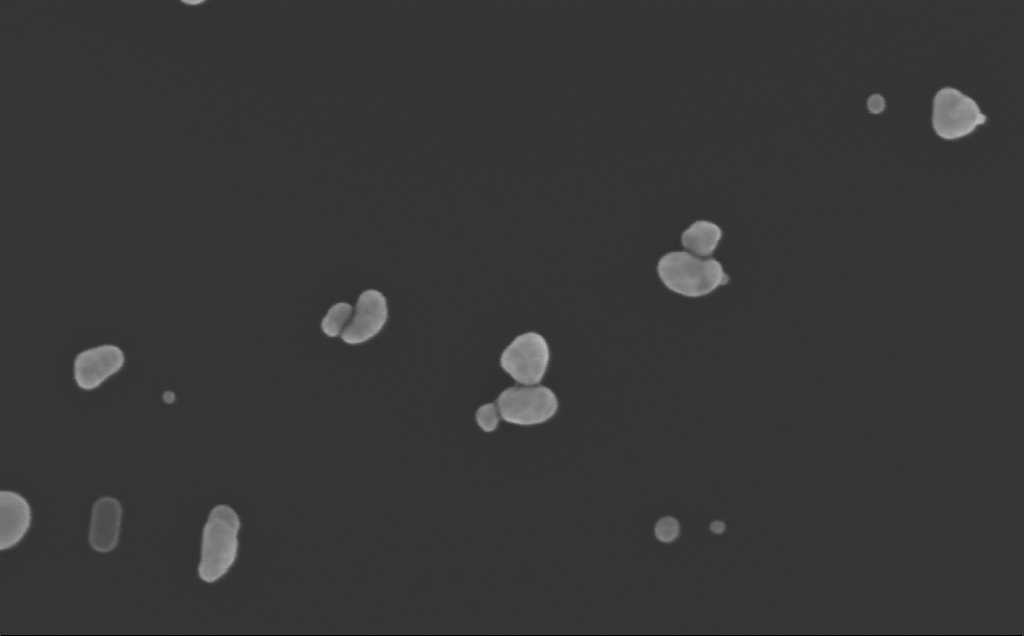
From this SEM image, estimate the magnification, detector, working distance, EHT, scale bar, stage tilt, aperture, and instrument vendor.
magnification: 250.48 K X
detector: InLens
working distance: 5 mm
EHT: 10 kV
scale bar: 200 nm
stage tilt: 0°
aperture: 30 µm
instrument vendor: Zeiss